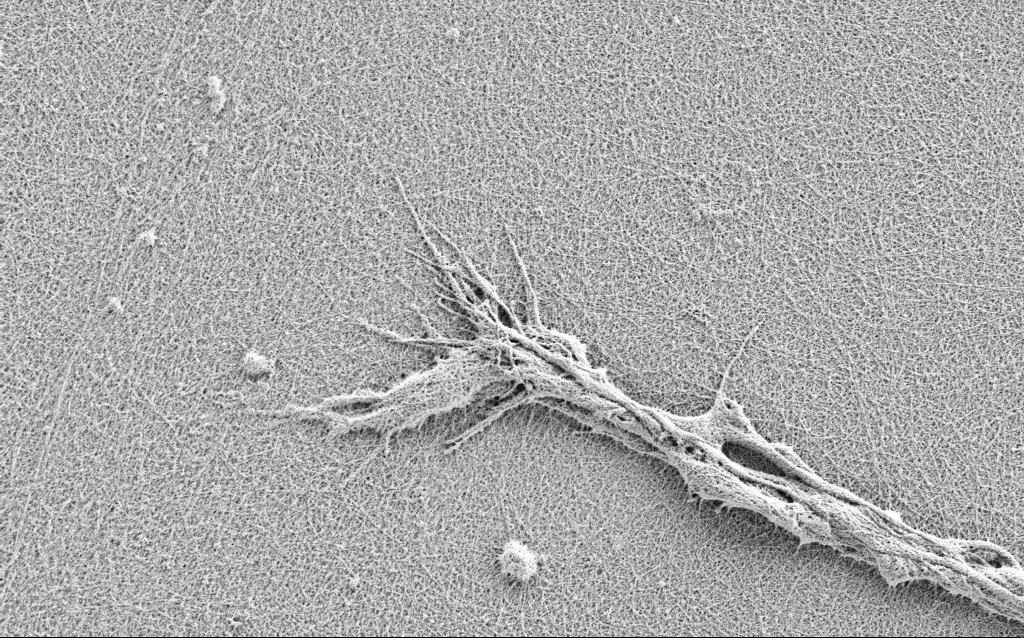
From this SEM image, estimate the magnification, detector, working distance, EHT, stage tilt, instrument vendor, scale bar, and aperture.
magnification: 10 K X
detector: SE2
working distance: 3 mm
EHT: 0.9 kV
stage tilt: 0°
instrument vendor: Zeiss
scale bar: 2000 nm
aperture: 30 µm